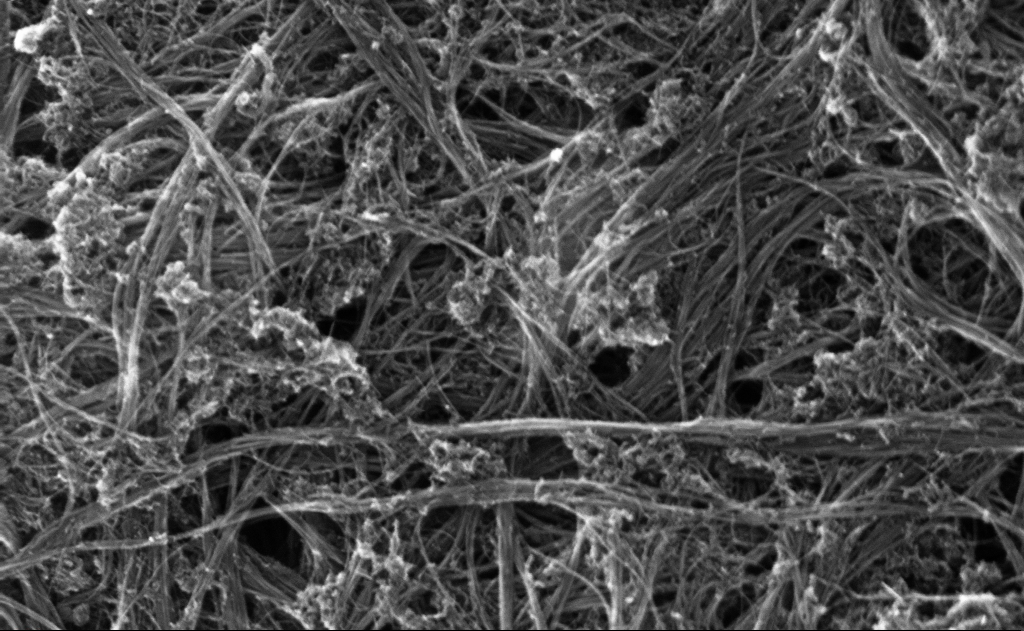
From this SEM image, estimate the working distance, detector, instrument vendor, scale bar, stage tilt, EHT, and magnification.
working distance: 6 mm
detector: InLens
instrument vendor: Zeiss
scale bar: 200 nm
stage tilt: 0°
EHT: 10 kV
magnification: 133.65 K X